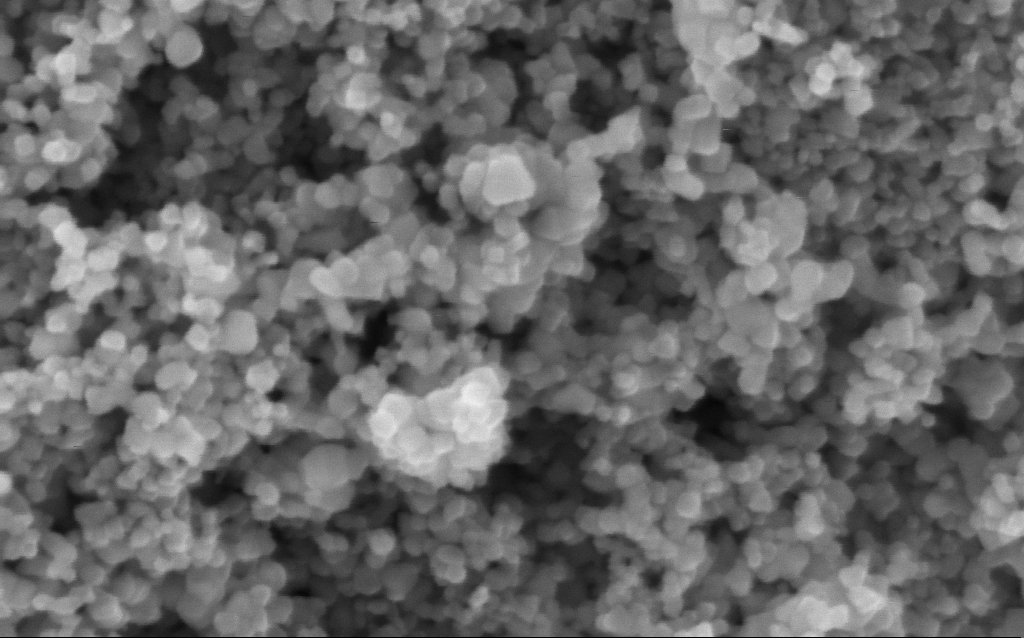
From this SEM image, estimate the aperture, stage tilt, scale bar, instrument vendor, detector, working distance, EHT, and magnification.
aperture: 30 µm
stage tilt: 0°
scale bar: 100 nm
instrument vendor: Zeiss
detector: InLens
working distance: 4.6 mm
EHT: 5 kV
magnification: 317 K X